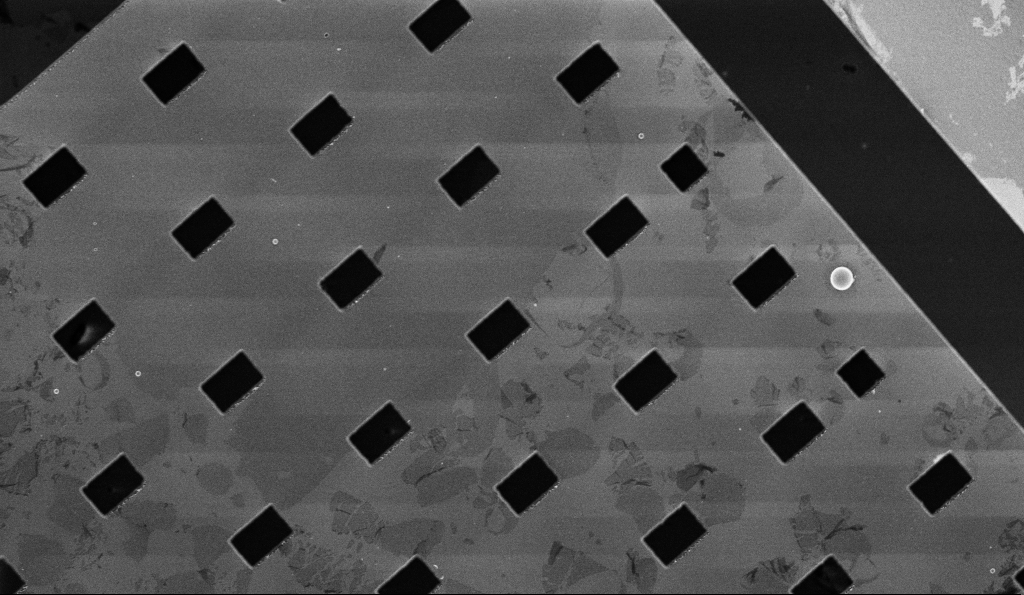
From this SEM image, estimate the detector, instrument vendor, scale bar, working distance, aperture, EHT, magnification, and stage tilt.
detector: InLens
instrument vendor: Zeiss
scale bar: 10000 nm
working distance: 5.8 mm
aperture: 30 µm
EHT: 3 kV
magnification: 4.75 K X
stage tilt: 0°